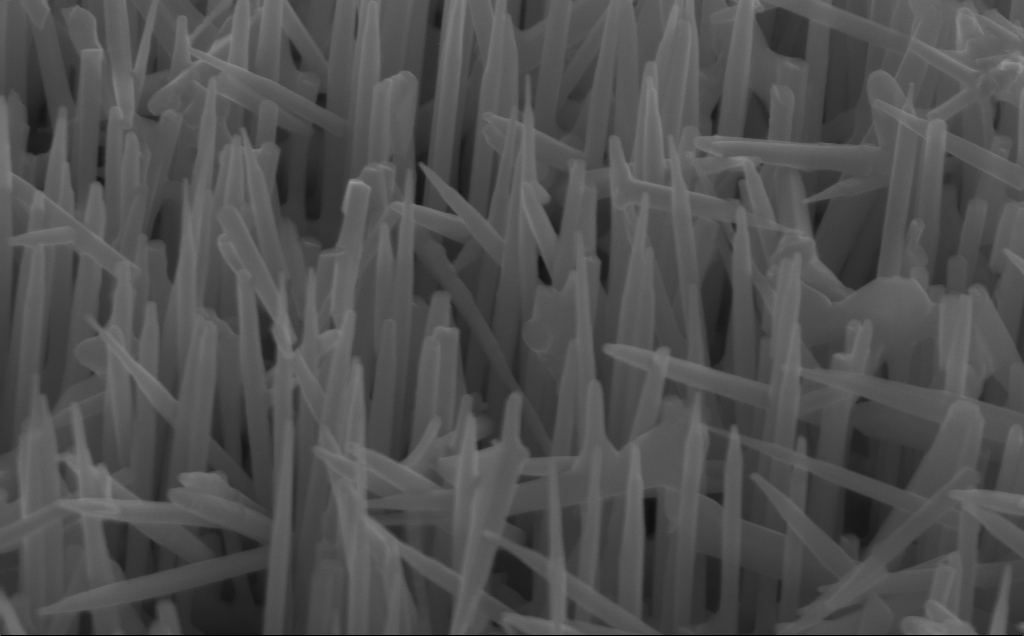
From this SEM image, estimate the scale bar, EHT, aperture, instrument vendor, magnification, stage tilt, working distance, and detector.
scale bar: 200 nm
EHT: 12 kV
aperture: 30 µm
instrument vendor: Zeiss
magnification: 80 K X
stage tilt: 45°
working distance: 5 mm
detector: InLens